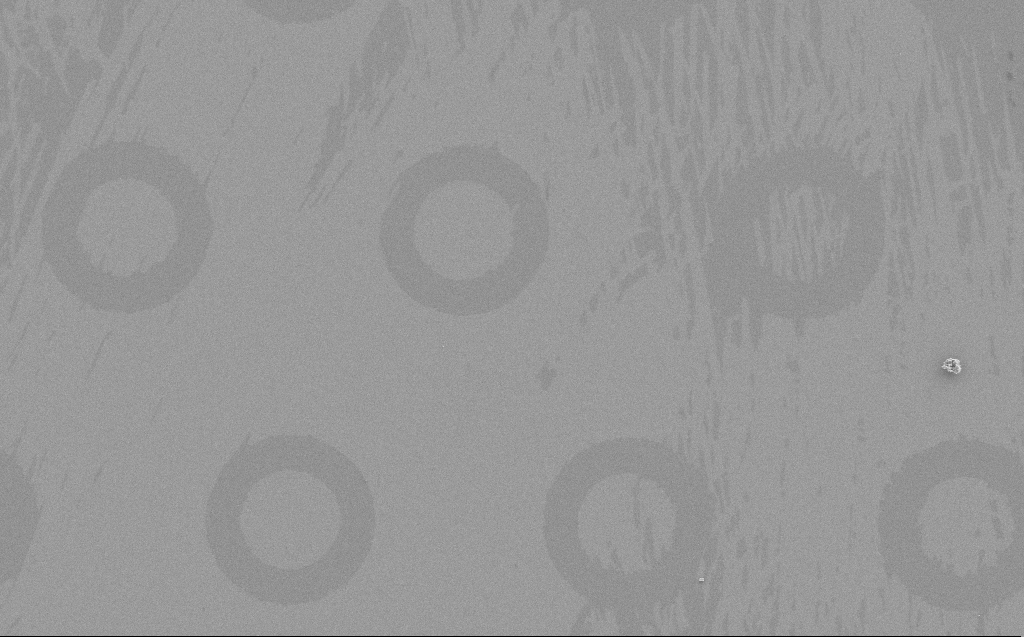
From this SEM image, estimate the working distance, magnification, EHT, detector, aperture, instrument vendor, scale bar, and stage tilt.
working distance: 6 mm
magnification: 1.25 K X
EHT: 5 kV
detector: SE2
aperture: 30 µm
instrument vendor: Zeiss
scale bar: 10000 nm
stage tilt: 0°